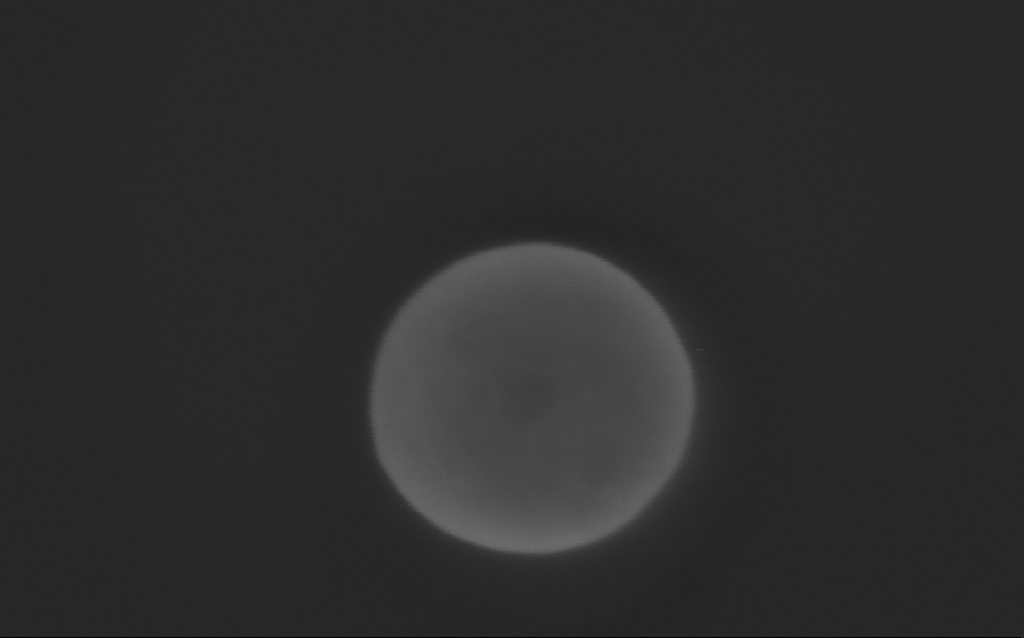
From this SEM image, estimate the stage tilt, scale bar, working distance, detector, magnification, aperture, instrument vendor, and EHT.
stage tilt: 0°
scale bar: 100 nm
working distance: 3 mm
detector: InLens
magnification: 449.87 K X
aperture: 30 µm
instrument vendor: Zeiss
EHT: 3 kV